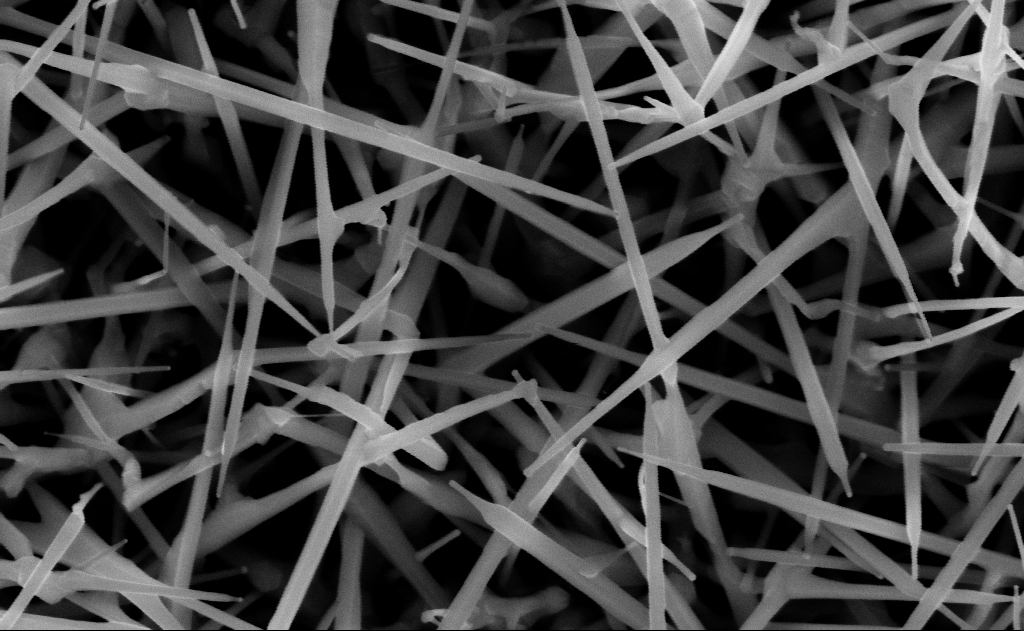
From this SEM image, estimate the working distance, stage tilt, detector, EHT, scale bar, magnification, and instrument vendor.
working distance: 13 mm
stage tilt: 0°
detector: InLens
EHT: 10 kV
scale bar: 200 nm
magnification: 80 K X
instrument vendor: Zeiss